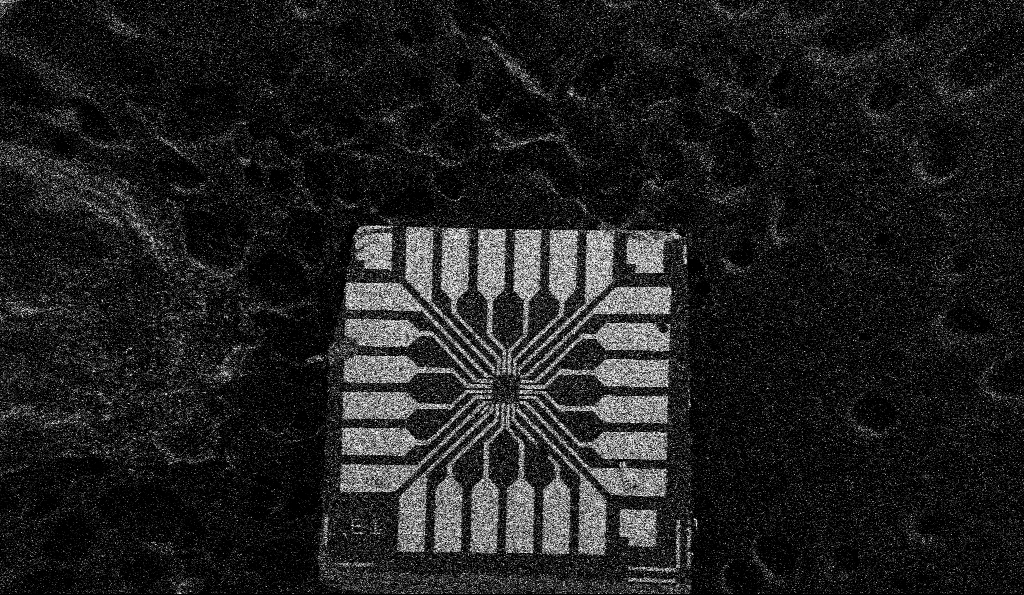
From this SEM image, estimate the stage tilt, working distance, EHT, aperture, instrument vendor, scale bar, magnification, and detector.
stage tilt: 0°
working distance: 8.5 mm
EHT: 5 kV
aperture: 30 µm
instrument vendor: Zeiss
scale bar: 1e+06 nm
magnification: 0.067 K X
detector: SE2